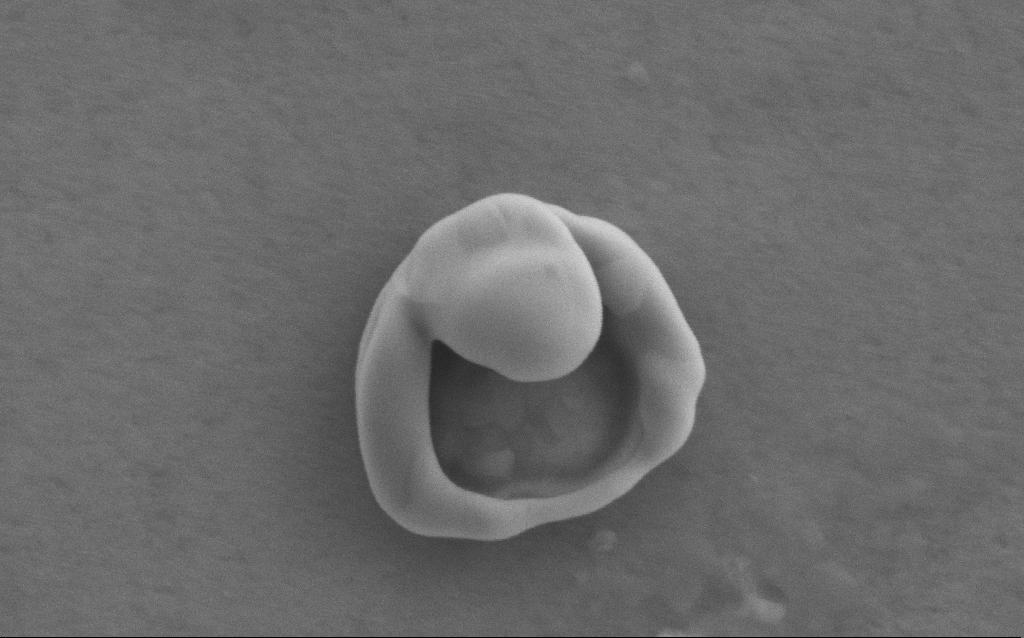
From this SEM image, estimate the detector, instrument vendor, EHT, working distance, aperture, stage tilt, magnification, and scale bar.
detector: SE2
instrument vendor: Zeiss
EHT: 5 kV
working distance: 4 mm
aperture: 30 µm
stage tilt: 0°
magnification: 120 K X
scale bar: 100 nm